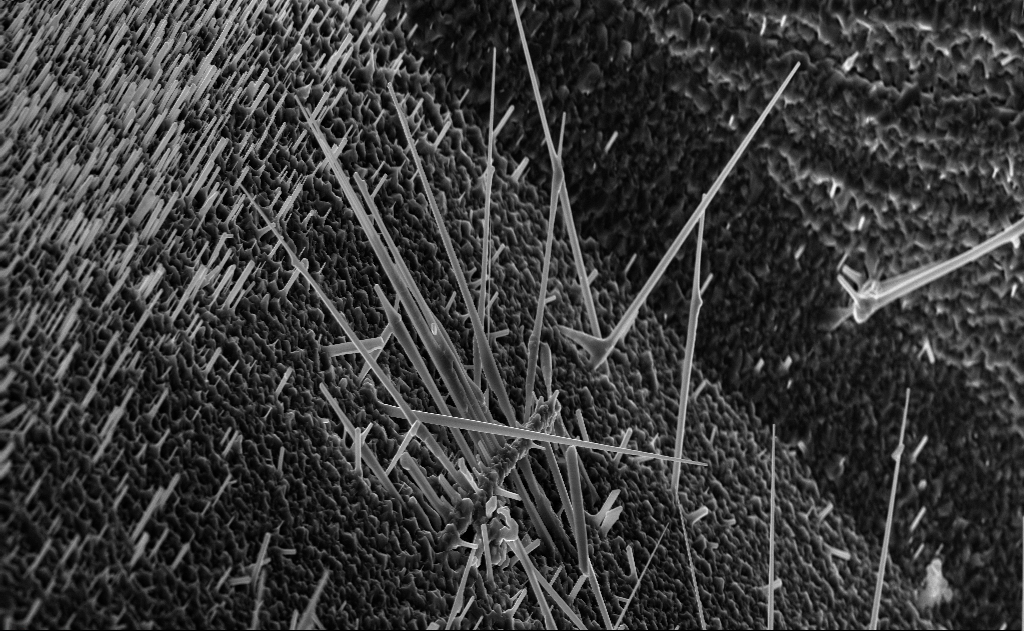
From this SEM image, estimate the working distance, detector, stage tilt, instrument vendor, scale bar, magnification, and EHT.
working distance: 5 mm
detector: InLens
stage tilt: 0°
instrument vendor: Zeiss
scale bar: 10000 nm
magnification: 7.12 K X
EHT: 10 kV